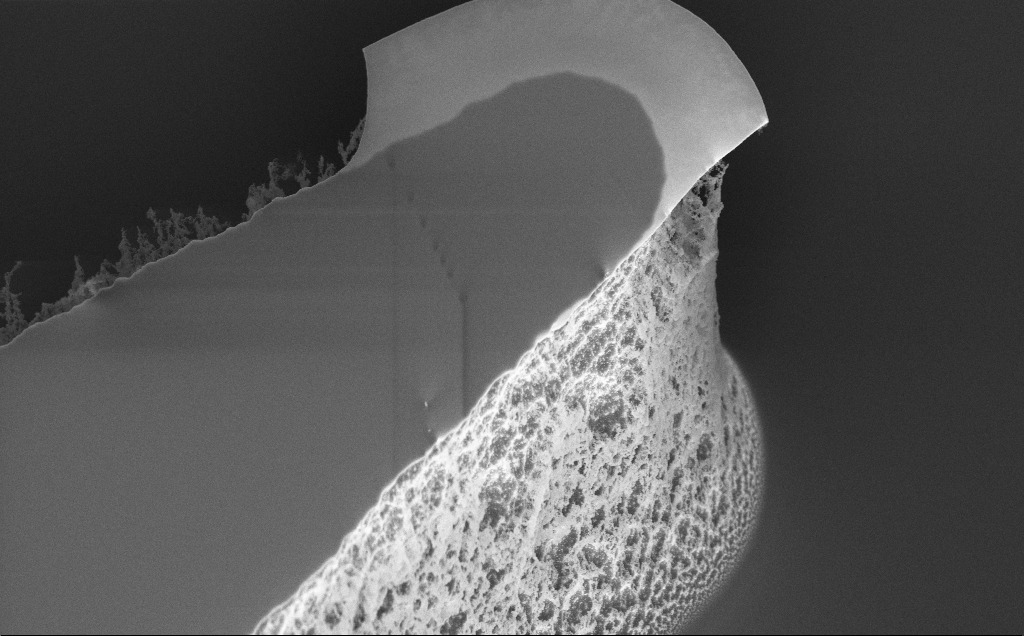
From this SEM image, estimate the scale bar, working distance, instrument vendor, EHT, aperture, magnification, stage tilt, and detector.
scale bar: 2000 nm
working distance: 8 mm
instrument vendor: Zeiss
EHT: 5 kV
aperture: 30 µm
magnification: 7.94 K X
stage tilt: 45°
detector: InLens